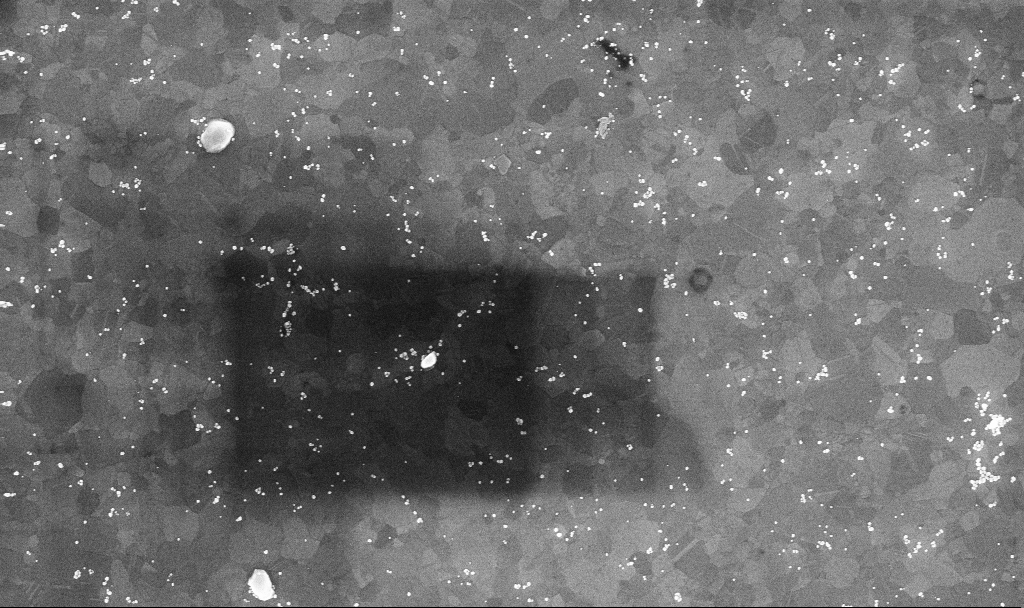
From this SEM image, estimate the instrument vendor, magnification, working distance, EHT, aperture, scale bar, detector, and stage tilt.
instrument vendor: Zeiss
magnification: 50.42 K X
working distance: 3.4 mm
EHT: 10 kV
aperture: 30 µm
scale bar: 1000 nm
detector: InLens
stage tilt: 0°